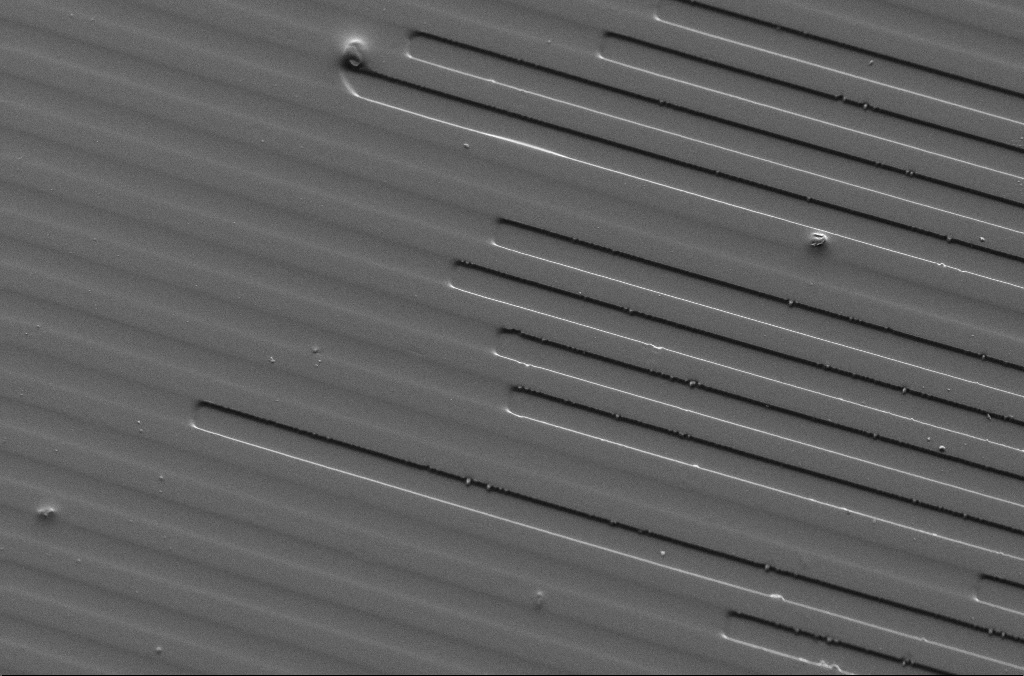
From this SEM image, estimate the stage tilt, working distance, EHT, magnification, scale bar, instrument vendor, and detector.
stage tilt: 40°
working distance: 7.2 mm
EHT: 5 kV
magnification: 1 K X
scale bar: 20000 nm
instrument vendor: Zeiss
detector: SE2